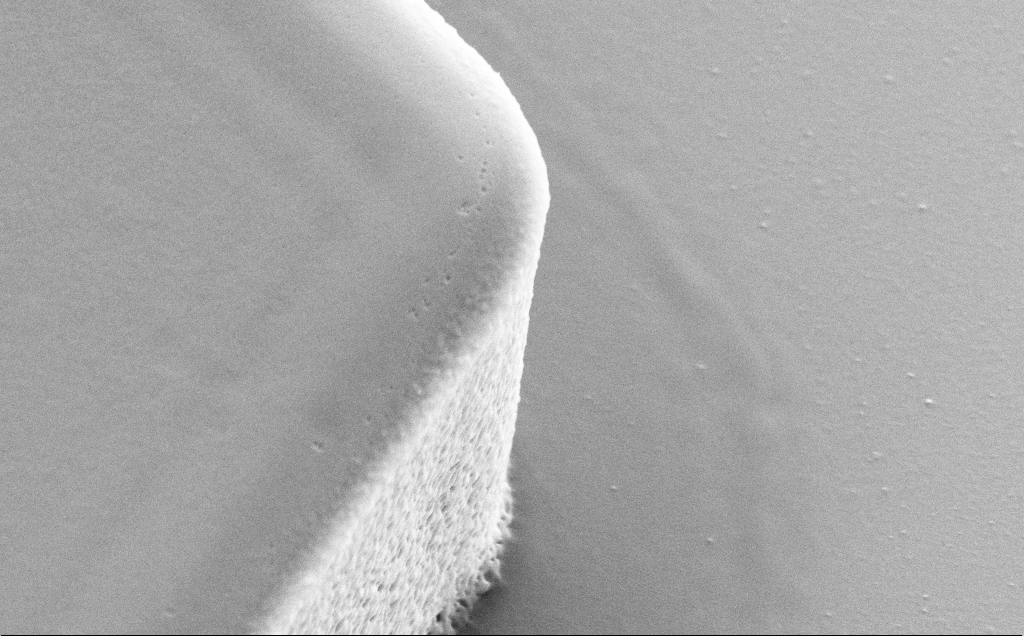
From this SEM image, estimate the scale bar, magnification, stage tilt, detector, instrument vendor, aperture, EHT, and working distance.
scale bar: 2000 nm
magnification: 26.11 K X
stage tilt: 30°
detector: SE2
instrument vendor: Zeiss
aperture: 30 µm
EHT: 5 kV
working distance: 9 mm